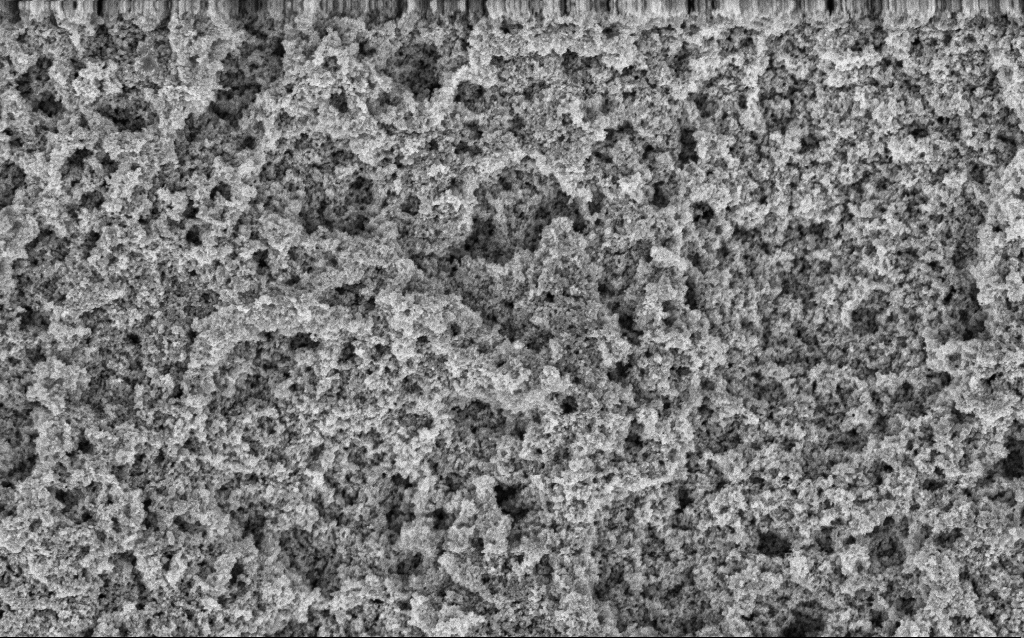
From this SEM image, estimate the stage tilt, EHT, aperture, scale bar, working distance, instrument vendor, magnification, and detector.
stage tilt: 0°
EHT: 3 kV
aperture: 30 µm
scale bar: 1000 nm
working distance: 10 mm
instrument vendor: Zeiss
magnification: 37.88 K X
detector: InLens